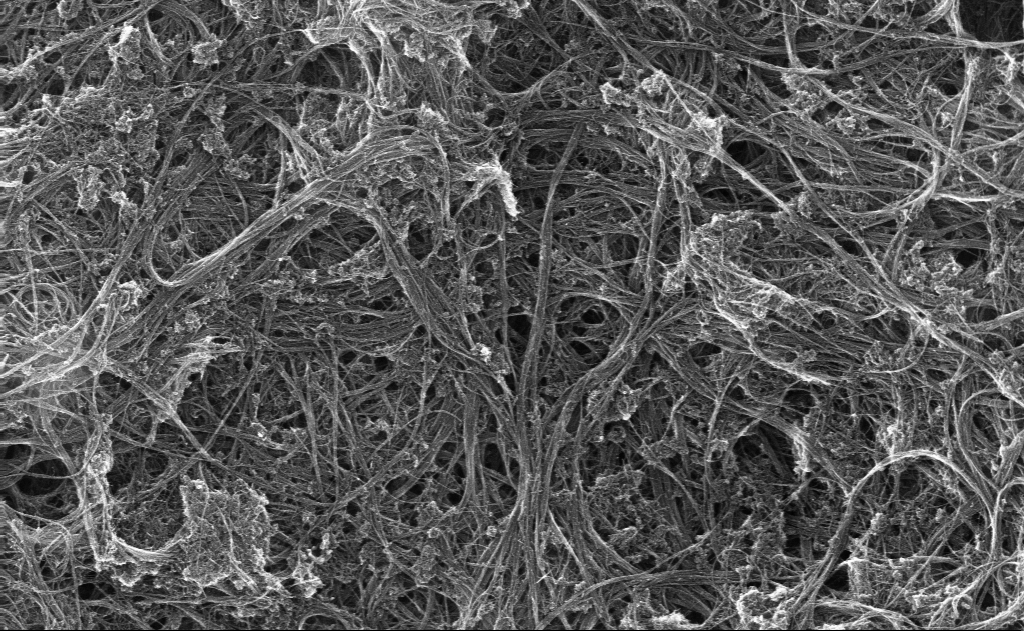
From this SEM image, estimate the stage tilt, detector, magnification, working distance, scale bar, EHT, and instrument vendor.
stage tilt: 0°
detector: InLens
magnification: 56.69 K X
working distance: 3 mm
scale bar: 1000 nm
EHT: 10 kV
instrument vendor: Zeiss